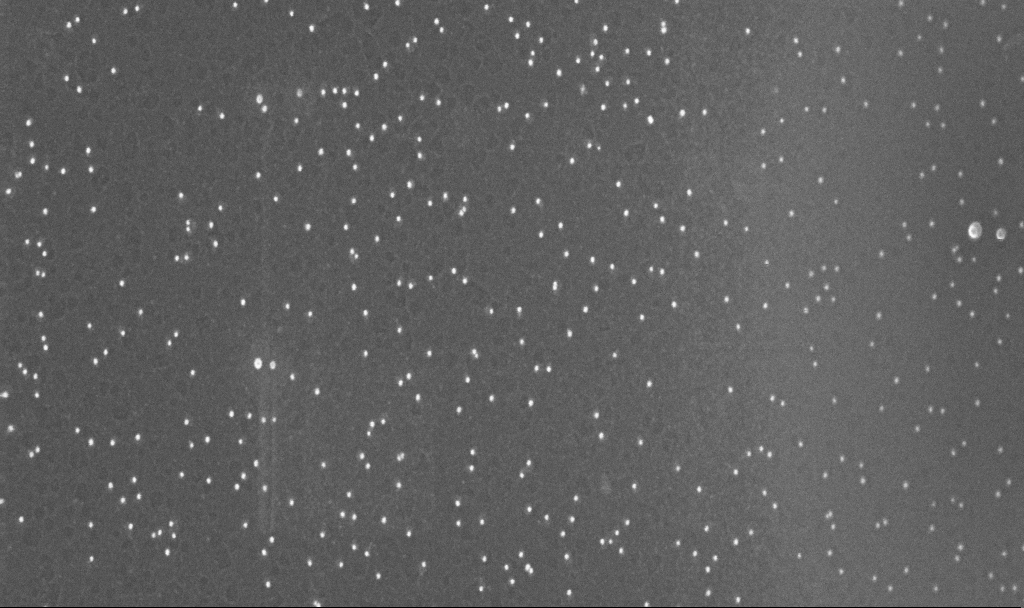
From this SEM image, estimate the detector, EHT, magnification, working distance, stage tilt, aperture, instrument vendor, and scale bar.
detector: InLens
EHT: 10 kV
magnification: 135.66 K X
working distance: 3.2 mm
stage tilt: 0°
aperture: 30 µm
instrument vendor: Zeiss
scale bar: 100 nm